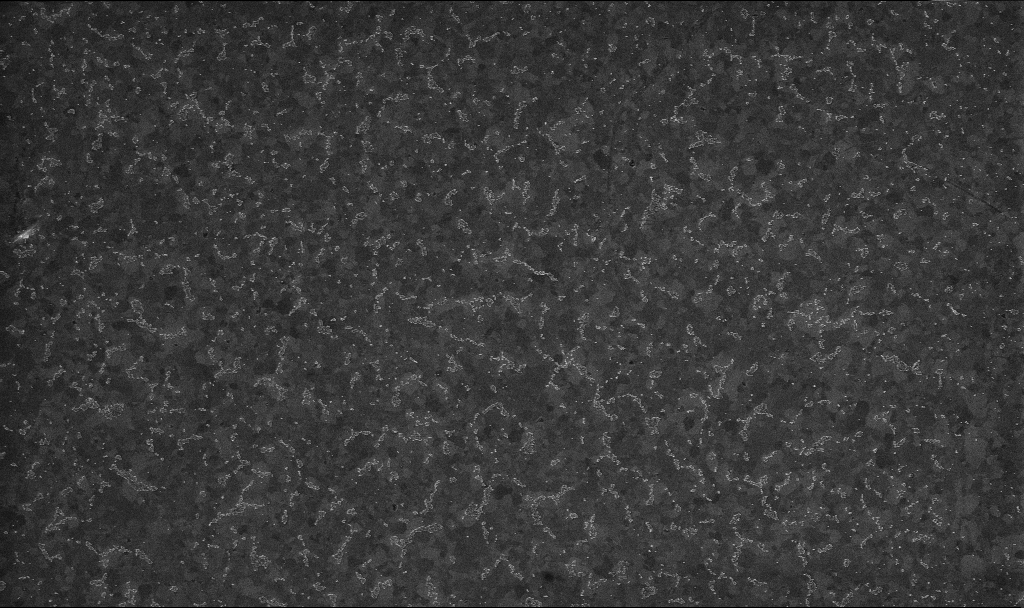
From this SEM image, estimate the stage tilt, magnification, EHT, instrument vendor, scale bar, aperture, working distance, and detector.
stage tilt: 0°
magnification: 20 K X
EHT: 10 kV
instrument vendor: Zeiss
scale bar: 1000 nm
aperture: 30 µm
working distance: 3.2 mm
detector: InLens